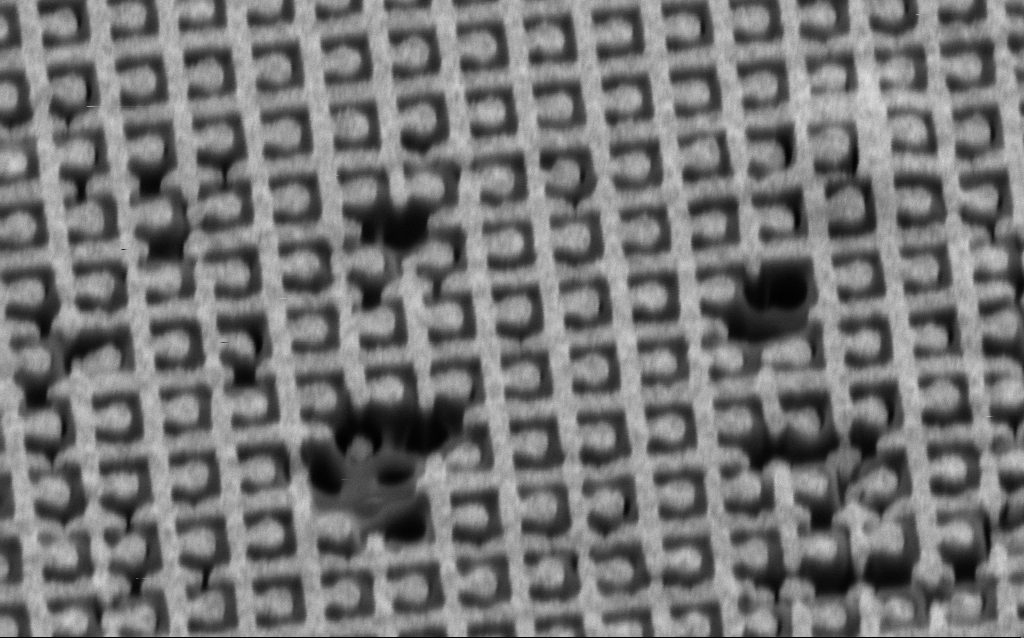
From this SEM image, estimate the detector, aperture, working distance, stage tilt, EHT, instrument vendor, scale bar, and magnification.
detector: SE2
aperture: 30 µm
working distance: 7.9 mm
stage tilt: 45°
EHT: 1.5 kV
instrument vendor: Zeiss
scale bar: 1000 nm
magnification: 54.34 K X